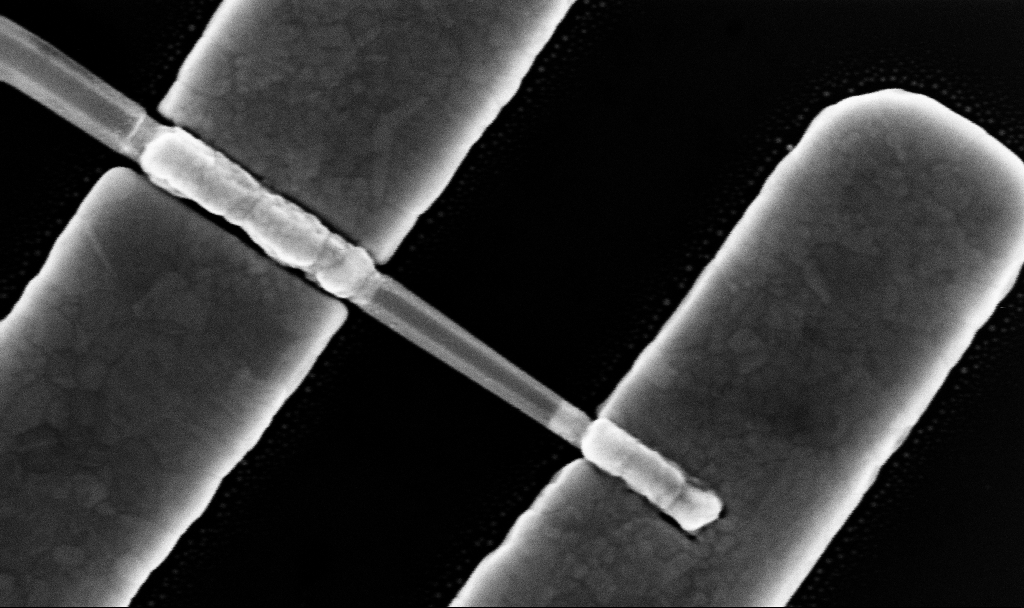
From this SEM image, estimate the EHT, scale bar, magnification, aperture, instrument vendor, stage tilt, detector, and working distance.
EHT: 10 kV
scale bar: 200 nm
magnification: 149.6 K X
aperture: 30 µm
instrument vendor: Zeiss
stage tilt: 0°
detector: InLens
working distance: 7.6 mm